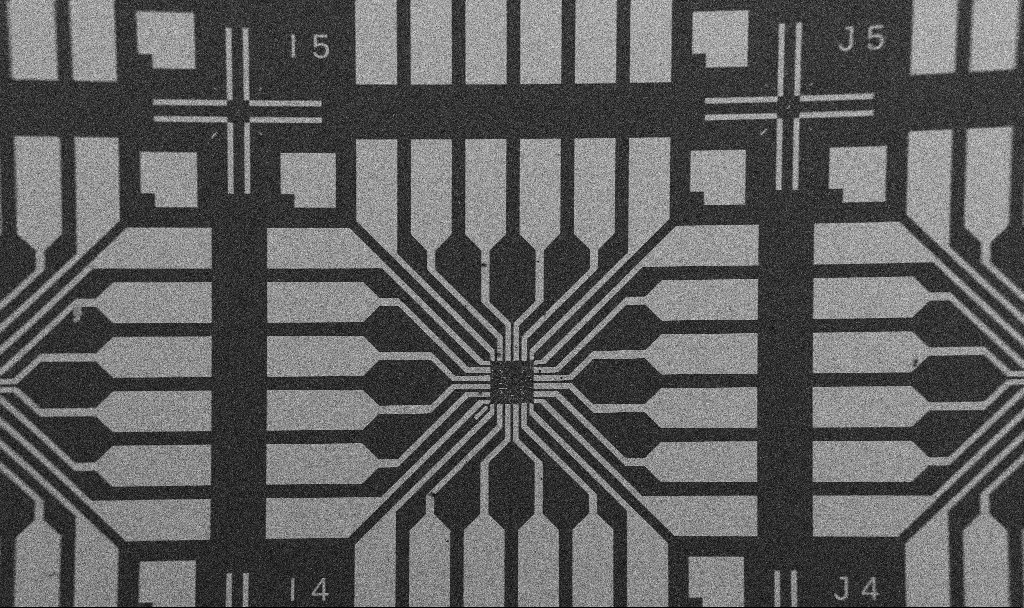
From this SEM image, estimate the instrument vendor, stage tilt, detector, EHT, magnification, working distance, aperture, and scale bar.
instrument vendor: Zeiss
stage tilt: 0°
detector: SE2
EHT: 5 kV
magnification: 0.1 K X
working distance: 10.7 mm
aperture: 30 µm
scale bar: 200000 nm